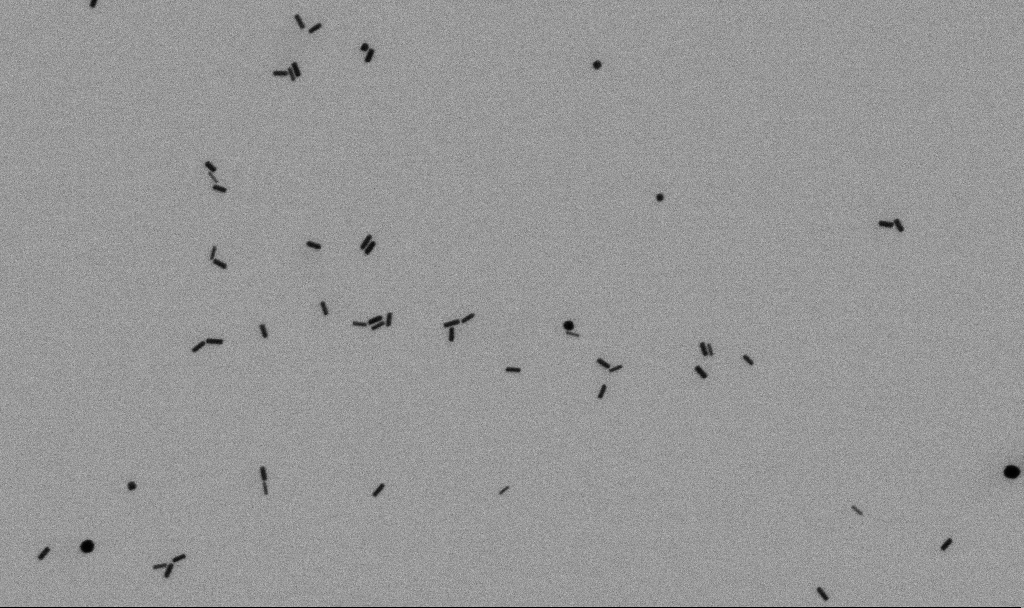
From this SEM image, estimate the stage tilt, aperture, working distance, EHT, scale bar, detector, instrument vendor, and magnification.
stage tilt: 0°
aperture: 30 µm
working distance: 4.5 mm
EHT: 5 kV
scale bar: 200 nm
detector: SE2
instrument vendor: Zeiss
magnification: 74.74 K X